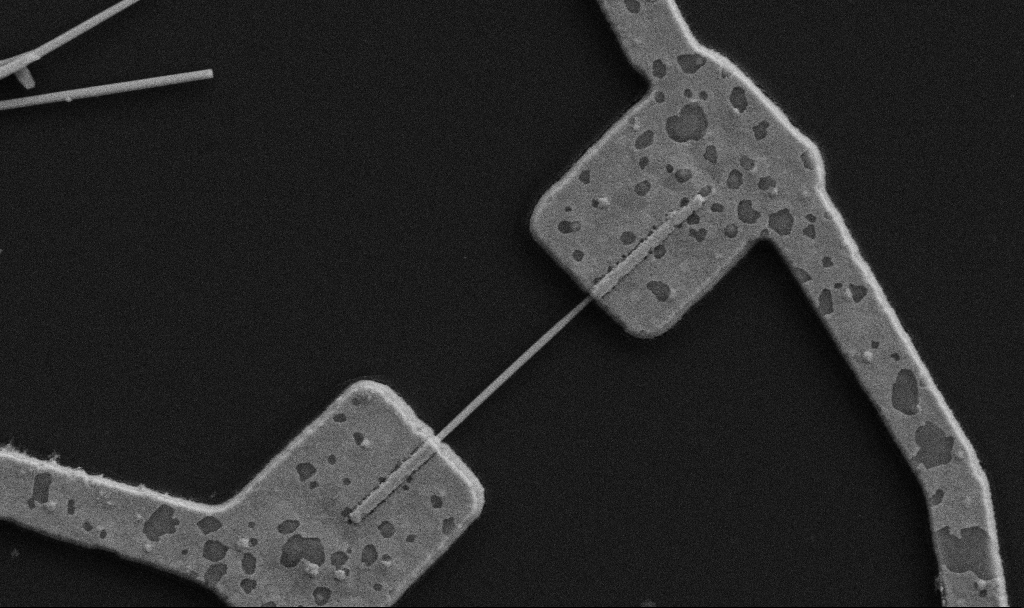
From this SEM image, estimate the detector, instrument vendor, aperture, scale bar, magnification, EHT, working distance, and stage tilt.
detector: SE2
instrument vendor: Zeiss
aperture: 30 µm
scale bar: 1000 nm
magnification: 30 K X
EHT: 5 kV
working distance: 8.7 mm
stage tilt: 0°